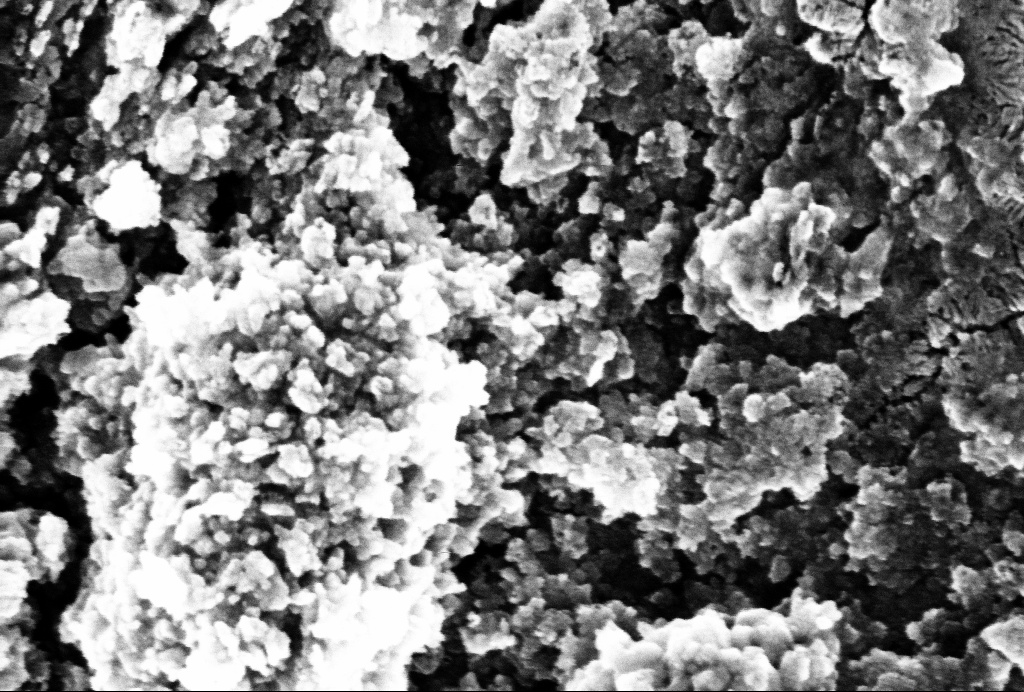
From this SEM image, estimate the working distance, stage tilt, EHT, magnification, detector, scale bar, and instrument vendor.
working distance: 2.7 mm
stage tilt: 45°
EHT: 5 kV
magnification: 200 K X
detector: InLens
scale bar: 200 nm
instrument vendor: Zeiss